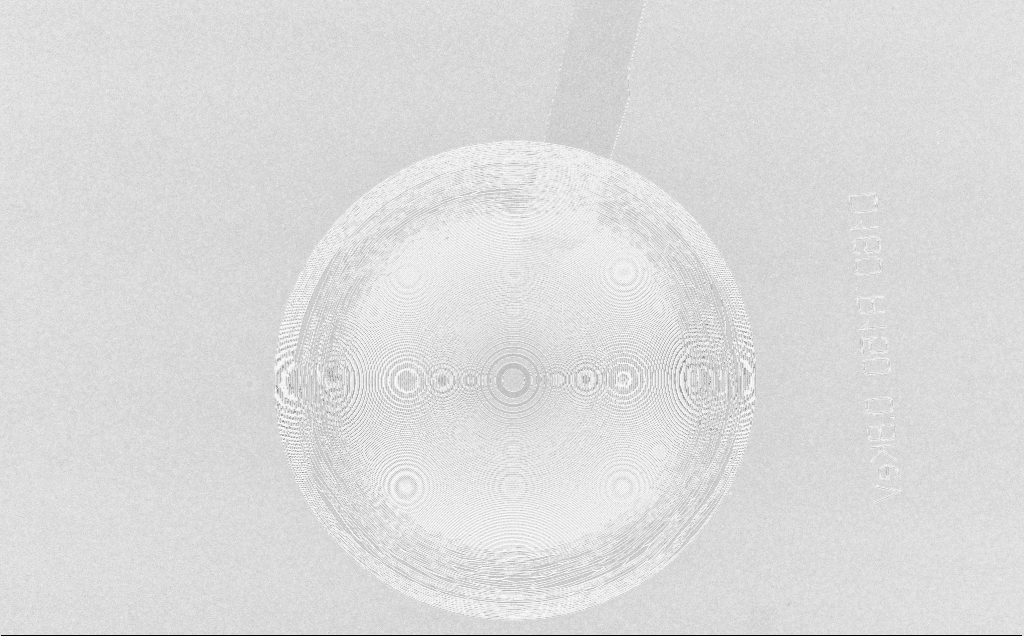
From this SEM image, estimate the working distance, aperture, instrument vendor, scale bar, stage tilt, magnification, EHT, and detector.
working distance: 6 mm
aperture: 30 µm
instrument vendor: Zeiss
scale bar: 20000 nm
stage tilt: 0°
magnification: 1.11 K X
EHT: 5 kV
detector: InLens